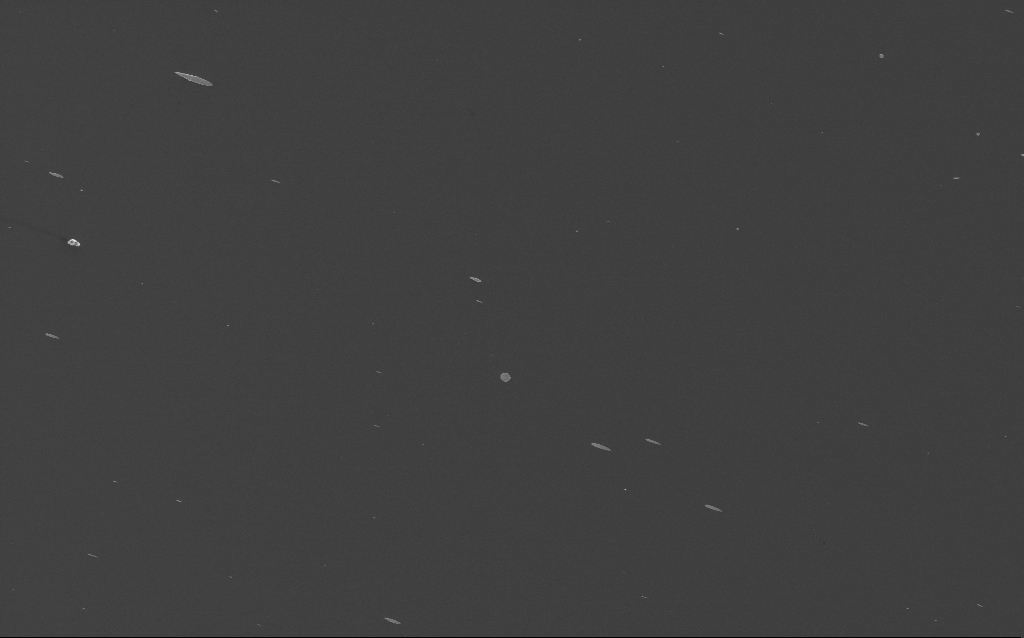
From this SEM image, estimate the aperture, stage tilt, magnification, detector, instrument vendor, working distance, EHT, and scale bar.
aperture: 30 µm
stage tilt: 0°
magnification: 3.87 K X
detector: InLens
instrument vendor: Zeiss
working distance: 3 mm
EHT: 3 kV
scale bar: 10000 nm